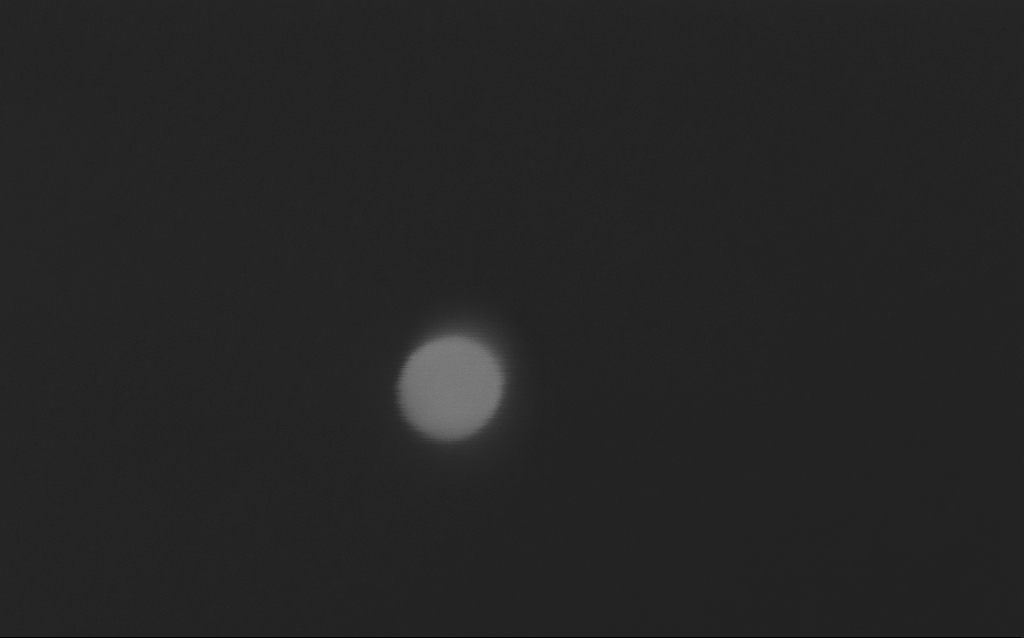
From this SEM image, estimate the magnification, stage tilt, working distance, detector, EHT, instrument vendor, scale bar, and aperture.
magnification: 735.89 K X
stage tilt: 0°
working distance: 3 mm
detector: InLens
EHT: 5 kV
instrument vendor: Zeiss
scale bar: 20 nm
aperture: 30 µm